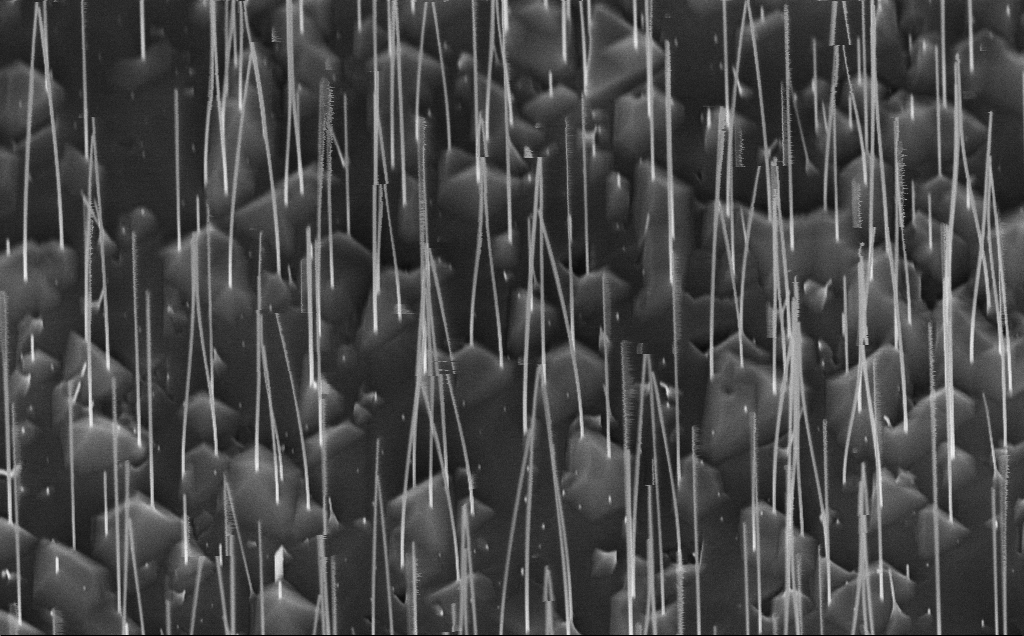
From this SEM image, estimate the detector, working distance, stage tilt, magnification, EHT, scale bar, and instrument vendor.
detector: InLens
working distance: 7 mm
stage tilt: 45°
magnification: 39.02 K X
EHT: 10 kV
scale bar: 1000 nm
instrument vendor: Zeiss